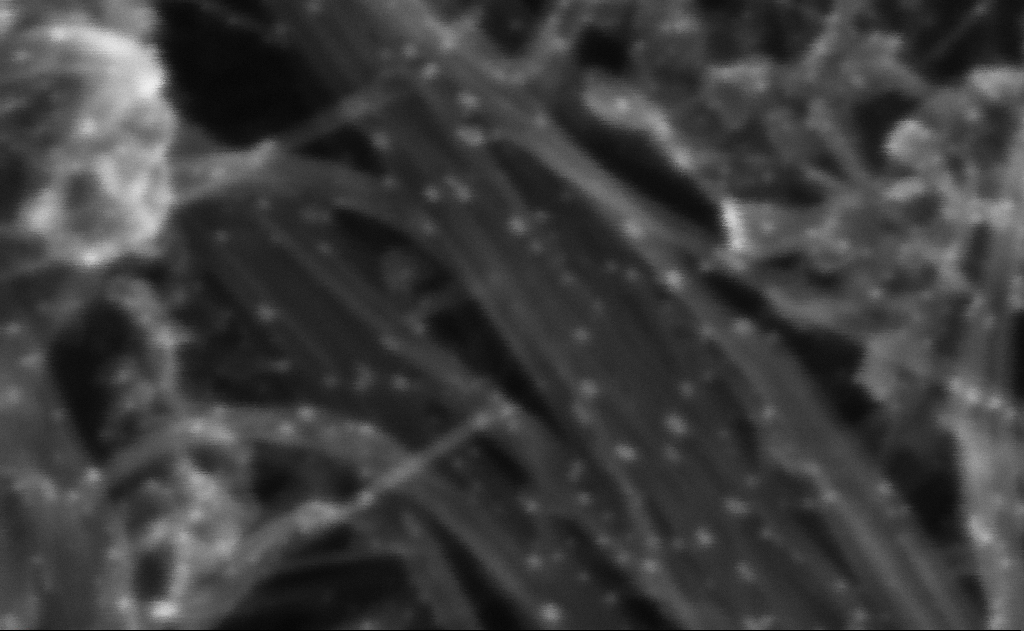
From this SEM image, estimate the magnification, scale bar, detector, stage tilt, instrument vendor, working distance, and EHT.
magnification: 1546.38 K X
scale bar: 20 nm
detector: InLens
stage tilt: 0°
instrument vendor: Zeiss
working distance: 3 mm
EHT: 10 kV